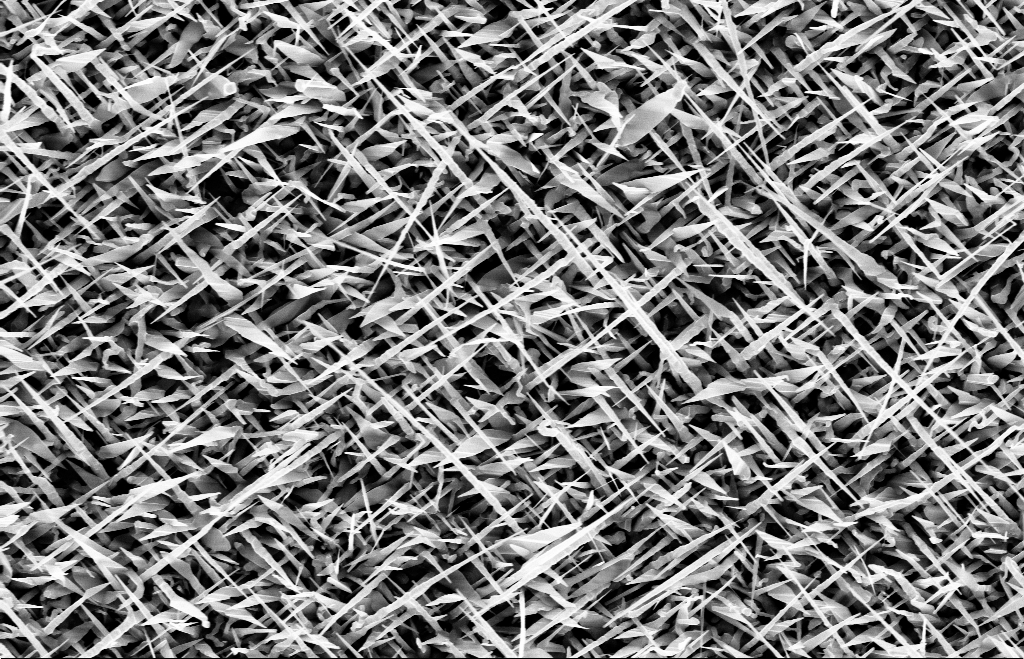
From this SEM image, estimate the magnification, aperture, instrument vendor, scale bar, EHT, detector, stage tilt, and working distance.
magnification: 20 K X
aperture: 30 µm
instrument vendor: Zeiss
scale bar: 1000 nm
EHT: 10 kV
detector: InLens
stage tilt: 0°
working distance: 8 mm